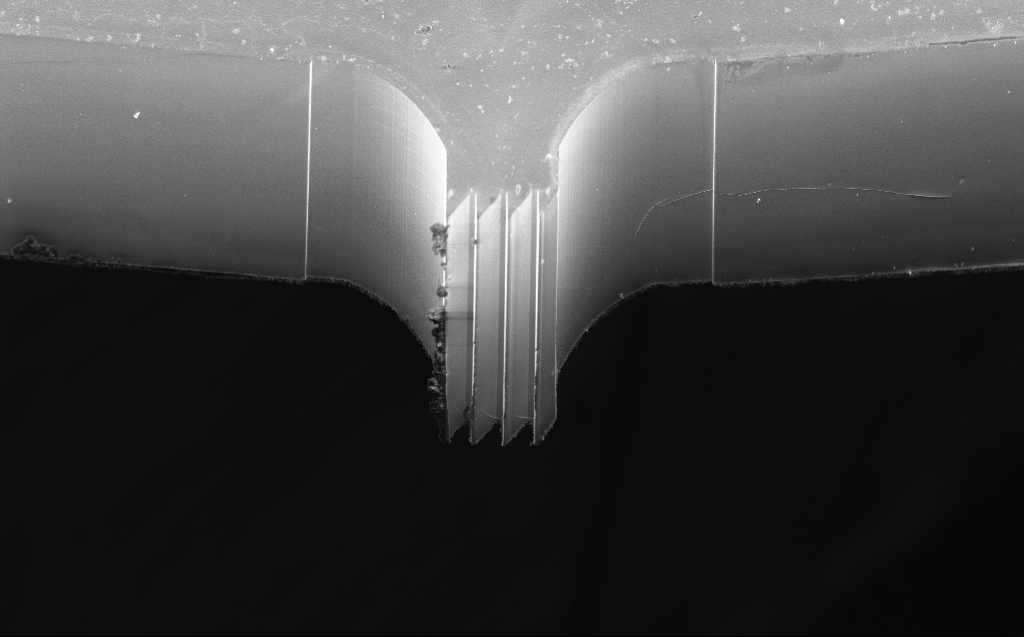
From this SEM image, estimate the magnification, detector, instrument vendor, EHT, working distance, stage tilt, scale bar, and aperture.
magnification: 1.01 K X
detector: InLens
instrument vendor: Zeiss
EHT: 5 kV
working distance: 5 mm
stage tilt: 45°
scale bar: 20000 nm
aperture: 30 µm